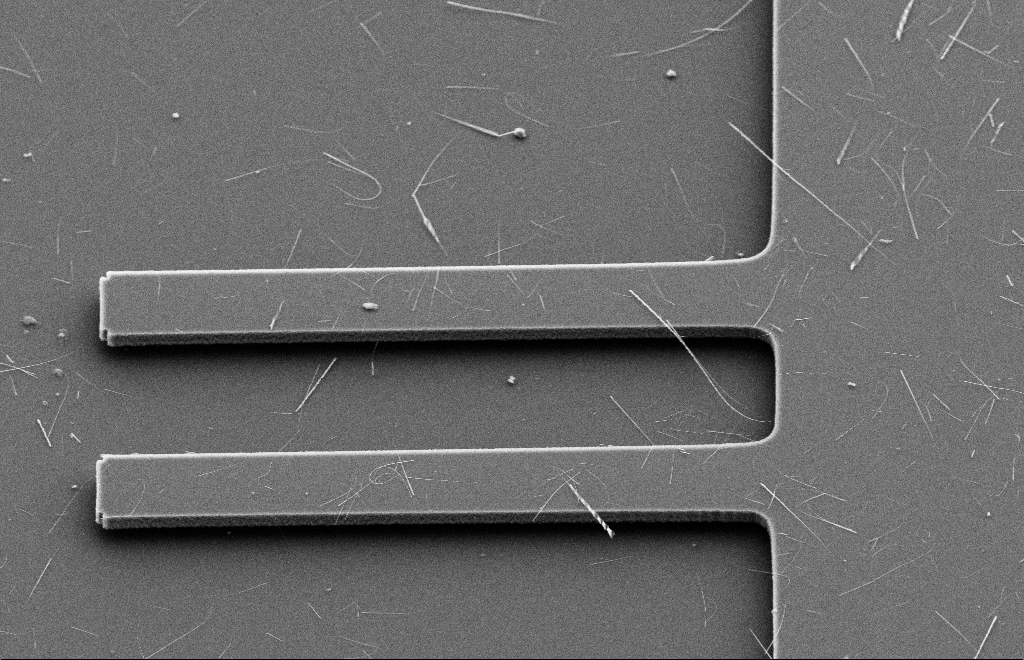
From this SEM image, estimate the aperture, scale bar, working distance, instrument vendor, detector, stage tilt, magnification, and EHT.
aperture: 20 µm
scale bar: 10000 nm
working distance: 9 mm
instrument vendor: Zeiss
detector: SE2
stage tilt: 39.8°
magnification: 2.5 K X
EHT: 10 kV